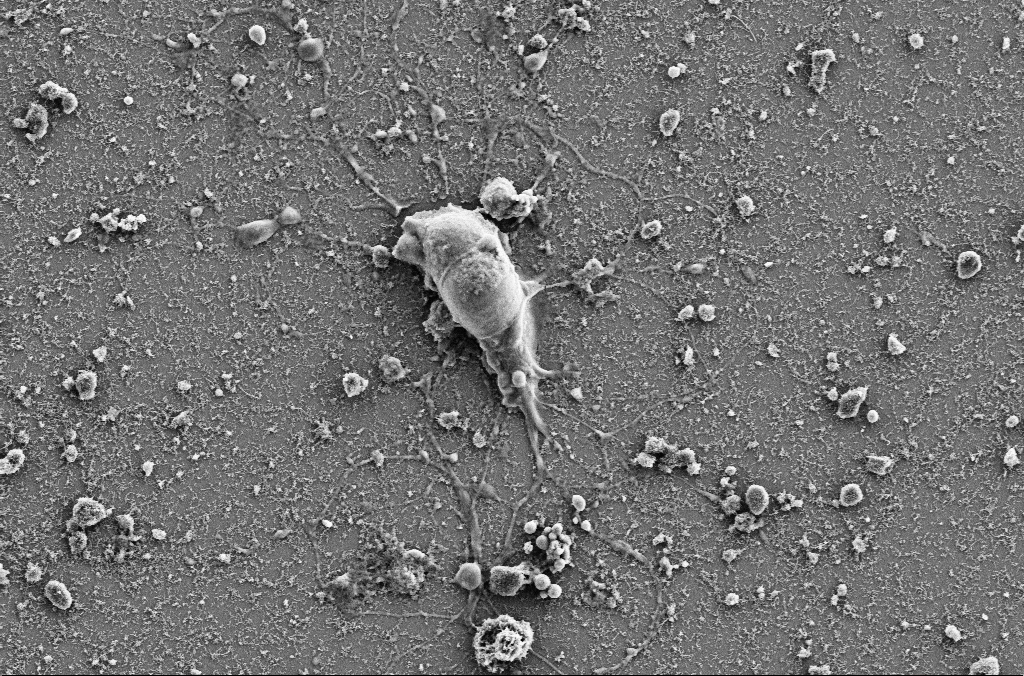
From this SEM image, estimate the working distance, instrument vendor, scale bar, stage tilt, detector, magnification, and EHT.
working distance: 4 mm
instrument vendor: Zeiss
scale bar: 10000 nm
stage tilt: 0°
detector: SE2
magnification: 4 K X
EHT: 5 kV